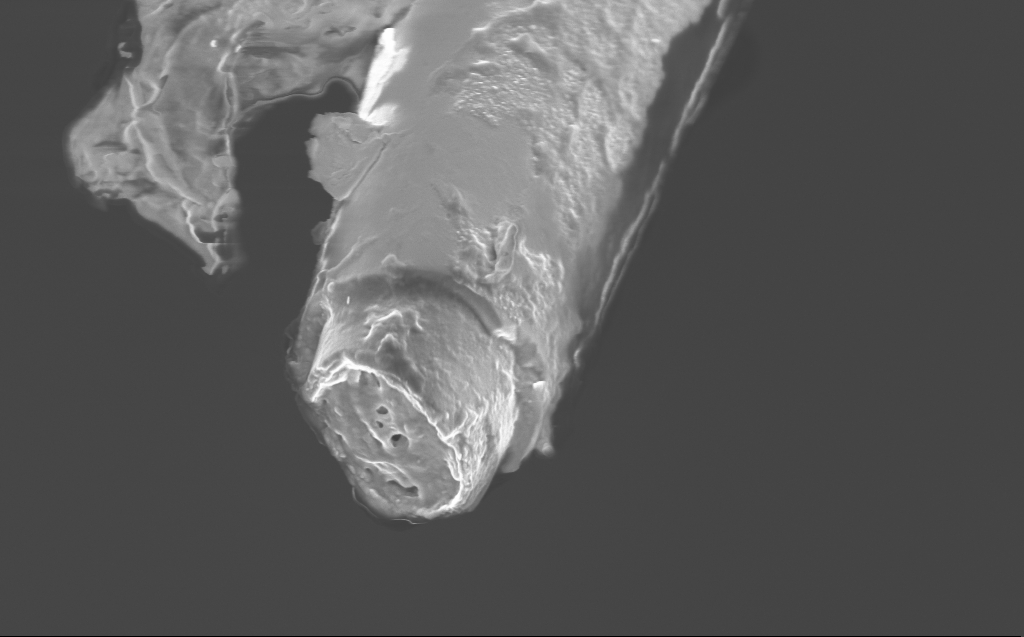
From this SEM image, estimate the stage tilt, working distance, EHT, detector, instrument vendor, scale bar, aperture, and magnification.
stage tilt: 45°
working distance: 2 mm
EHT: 2 kV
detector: InLens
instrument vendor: Zeiss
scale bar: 2000 nm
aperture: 30 µm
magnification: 29.93 K X